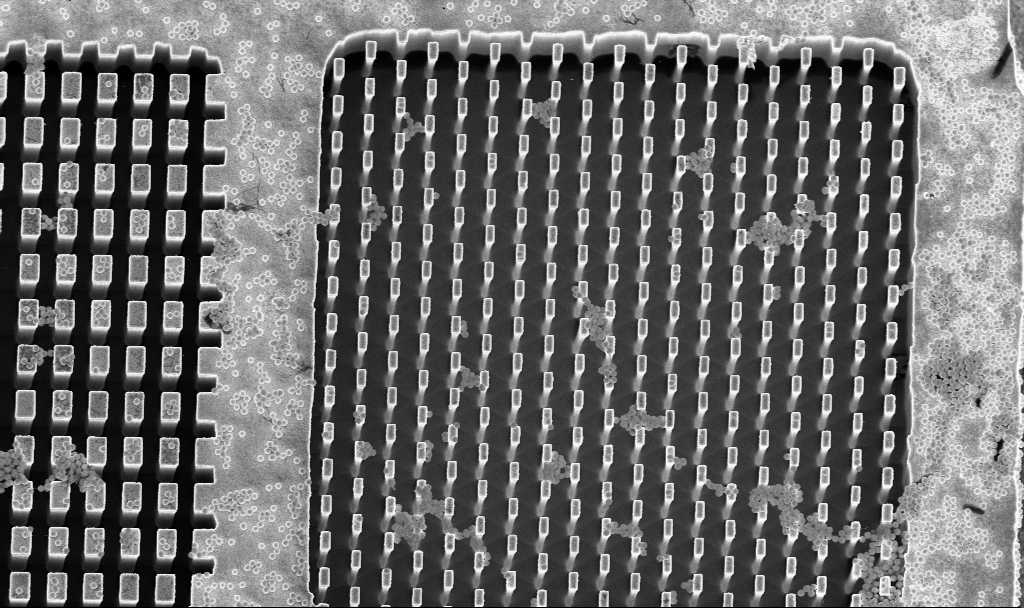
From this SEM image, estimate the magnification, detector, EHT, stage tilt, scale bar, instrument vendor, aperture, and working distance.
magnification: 4.19 K X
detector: InLens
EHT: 5 kV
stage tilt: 8°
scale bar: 10000 nm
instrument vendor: Zeiss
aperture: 30 µm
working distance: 3.3 mm